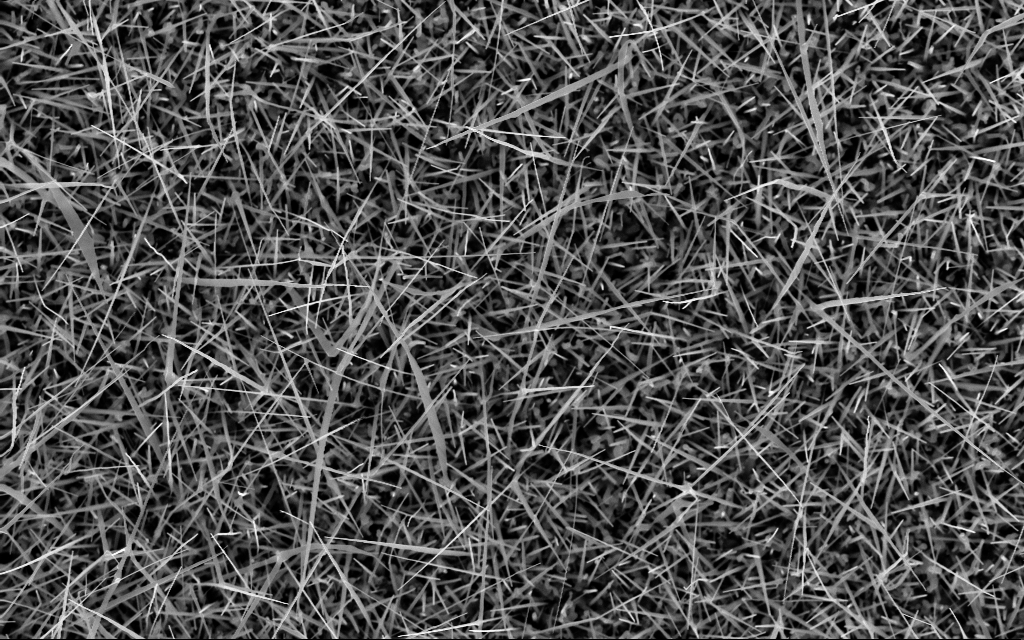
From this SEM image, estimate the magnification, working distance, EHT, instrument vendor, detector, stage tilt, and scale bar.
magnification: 20 K X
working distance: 5 mm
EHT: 10 kV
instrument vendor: Zeiss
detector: InLens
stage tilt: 0°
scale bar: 1000 nm